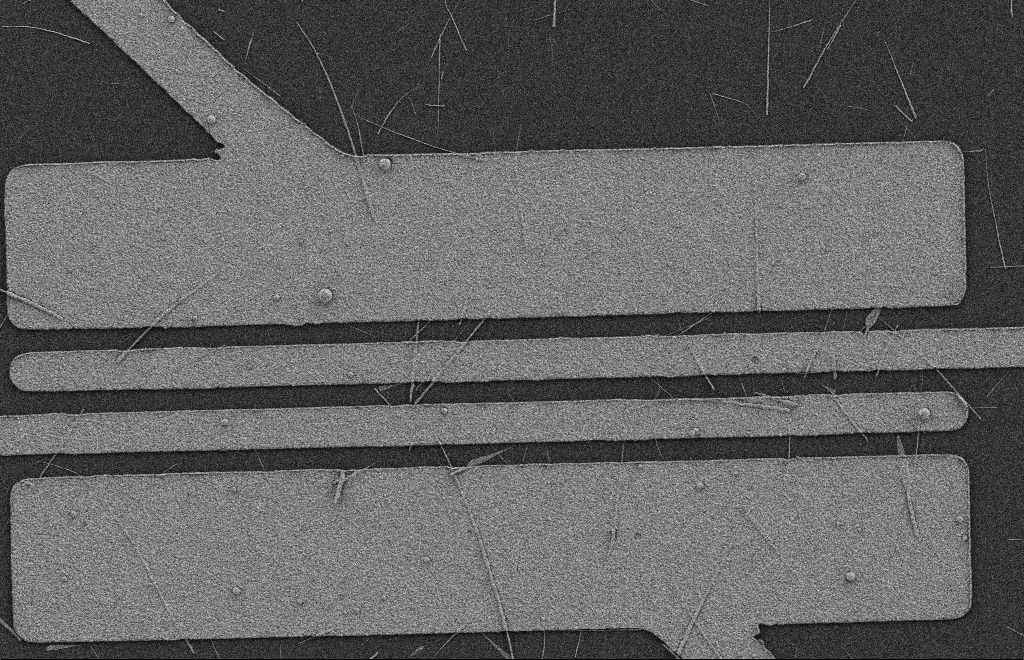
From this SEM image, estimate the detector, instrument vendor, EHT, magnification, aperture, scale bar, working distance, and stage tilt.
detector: SE2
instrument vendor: Zeiss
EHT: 2 kV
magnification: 5.75 K X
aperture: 20 µm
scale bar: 2000 nm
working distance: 8 mm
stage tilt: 0°